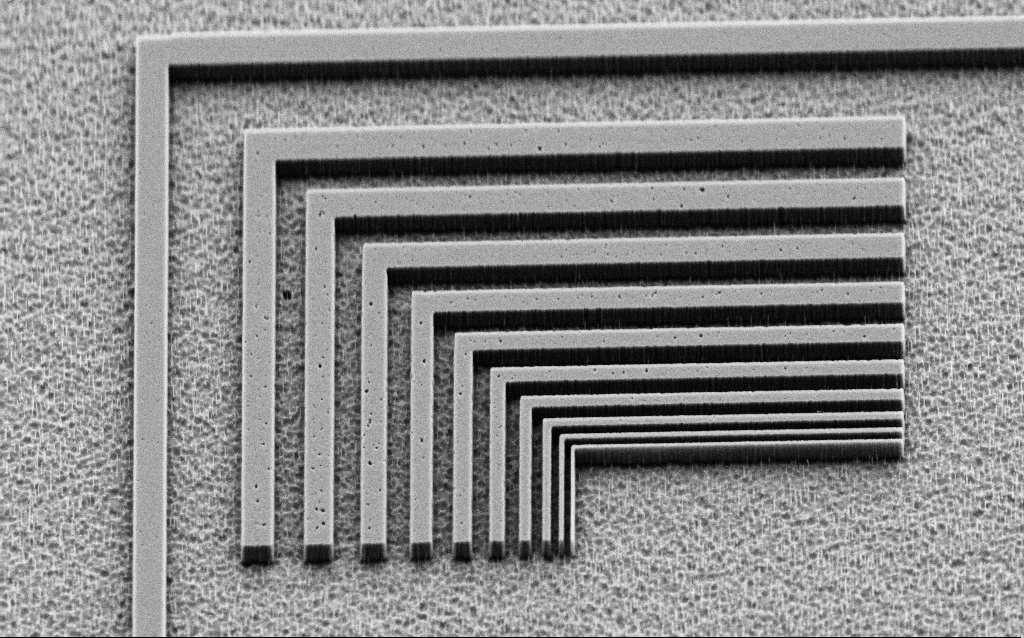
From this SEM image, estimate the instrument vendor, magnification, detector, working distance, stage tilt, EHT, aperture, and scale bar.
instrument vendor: Zeiss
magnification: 12.28 K X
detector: SE2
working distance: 5 mm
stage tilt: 45°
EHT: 3 kV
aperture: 30 µm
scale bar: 1000 nm